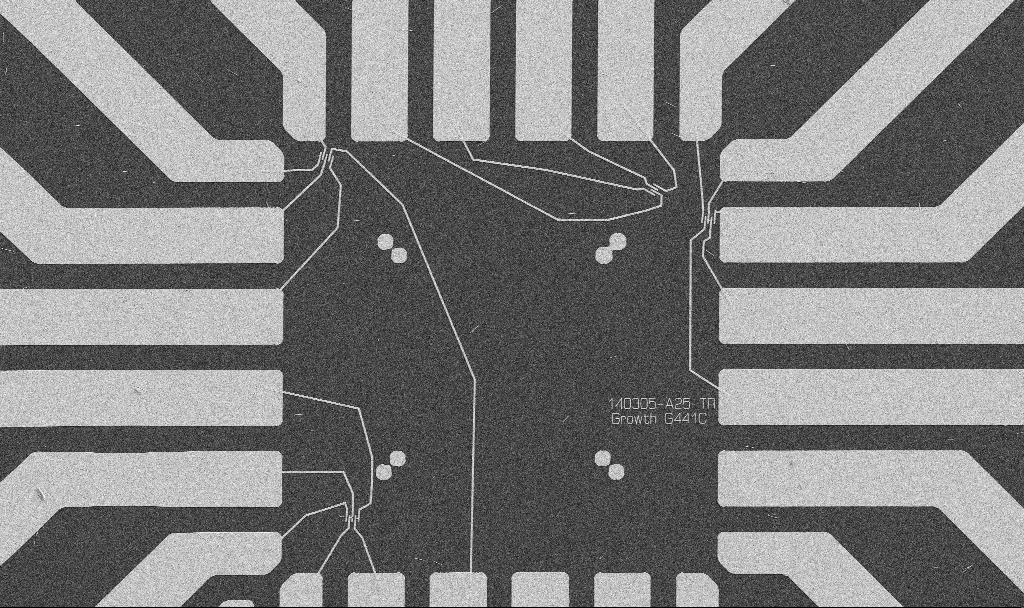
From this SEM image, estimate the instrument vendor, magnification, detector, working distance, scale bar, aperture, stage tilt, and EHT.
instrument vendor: Zeiss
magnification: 1 K X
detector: SE2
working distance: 10.7 mm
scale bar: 20000 nm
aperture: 30 µm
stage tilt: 0°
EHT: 5 kV